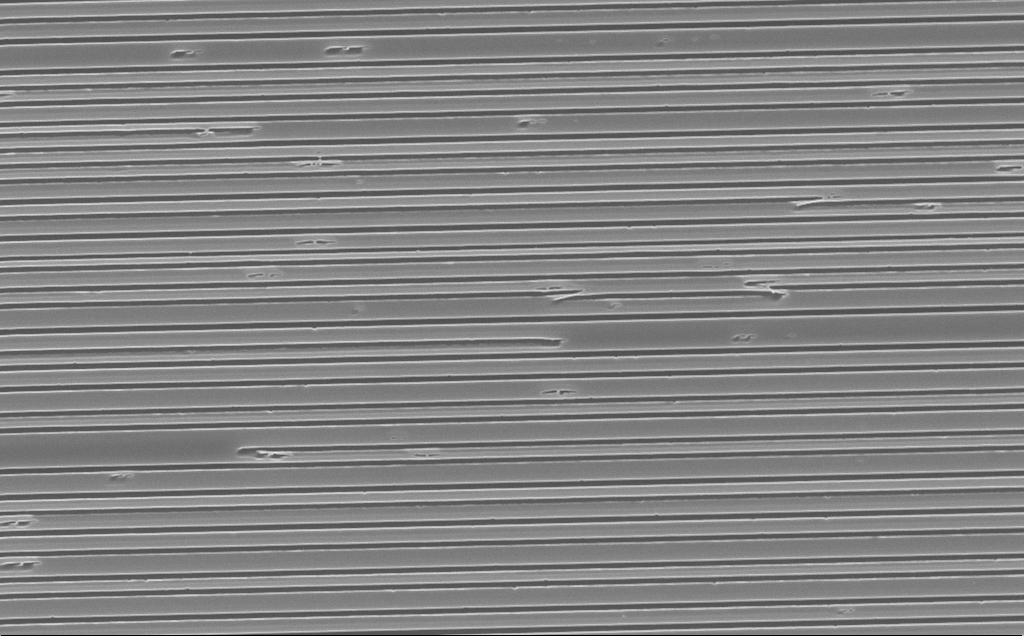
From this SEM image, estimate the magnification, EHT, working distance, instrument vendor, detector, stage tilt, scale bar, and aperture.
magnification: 9.58 K X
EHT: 10 kV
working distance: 4 mm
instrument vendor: Zeiss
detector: InLens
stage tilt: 0°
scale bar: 2000 nm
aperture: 30 µm